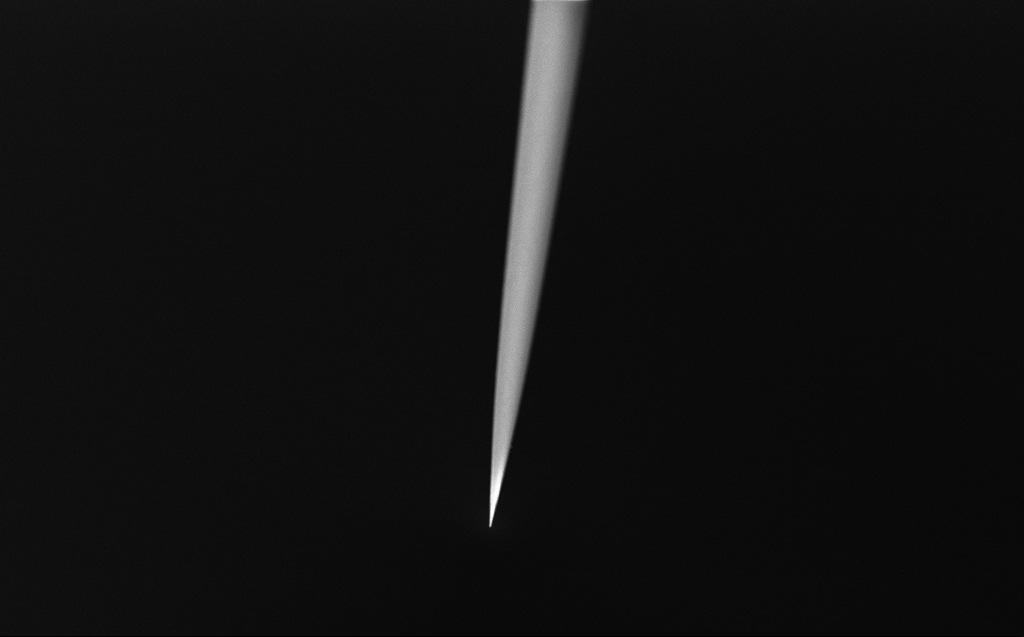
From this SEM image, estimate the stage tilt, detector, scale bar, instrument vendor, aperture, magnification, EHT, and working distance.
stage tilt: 45°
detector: InLens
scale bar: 100000 nm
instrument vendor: Zeiss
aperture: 30 µm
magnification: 0.692 K X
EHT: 2 kV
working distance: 3 mm